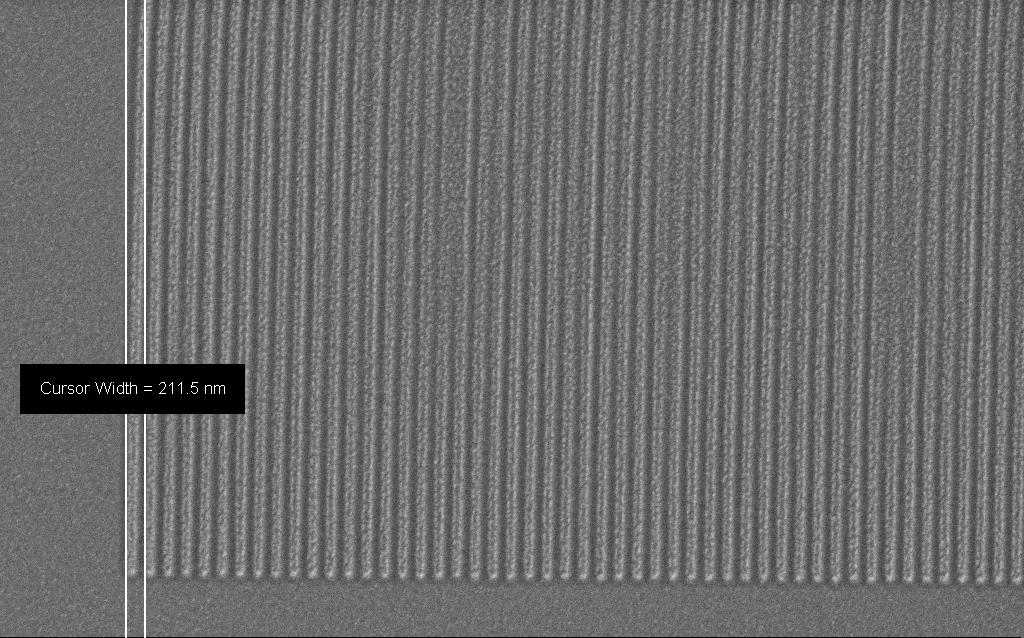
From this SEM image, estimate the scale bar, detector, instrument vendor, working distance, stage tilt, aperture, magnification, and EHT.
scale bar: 2000 nm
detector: SE2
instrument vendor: Zeiss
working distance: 6 mm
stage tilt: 0°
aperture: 30 µm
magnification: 32.98 K X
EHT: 1.5 kV